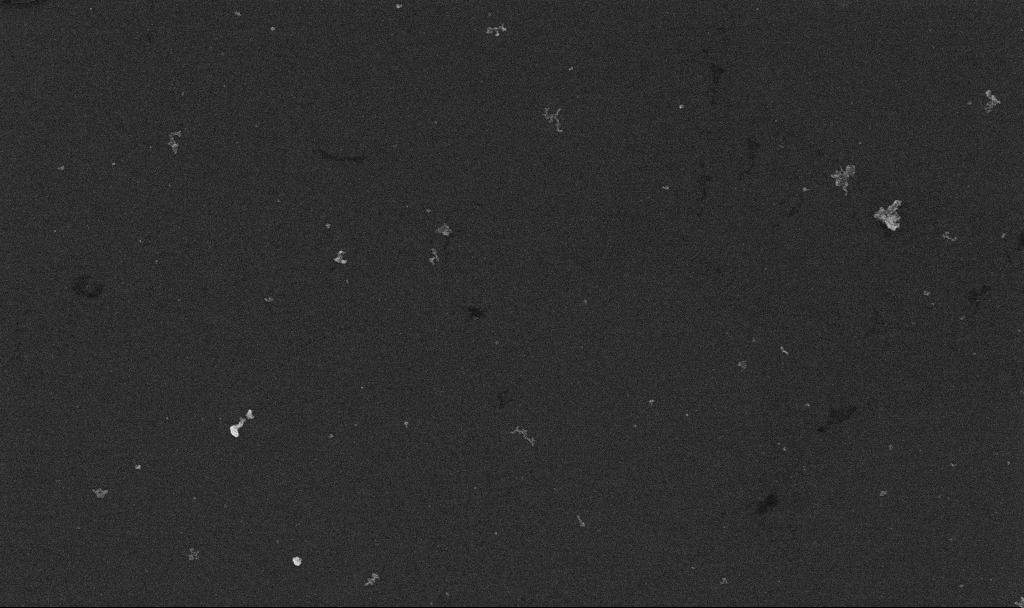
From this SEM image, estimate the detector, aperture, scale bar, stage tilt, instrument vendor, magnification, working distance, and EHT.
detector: InLens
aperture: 30 µm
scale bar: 2000 nm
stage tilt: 0°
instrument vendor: Zeiss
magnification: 10 K X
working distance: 3.3 mm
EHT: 10 kV